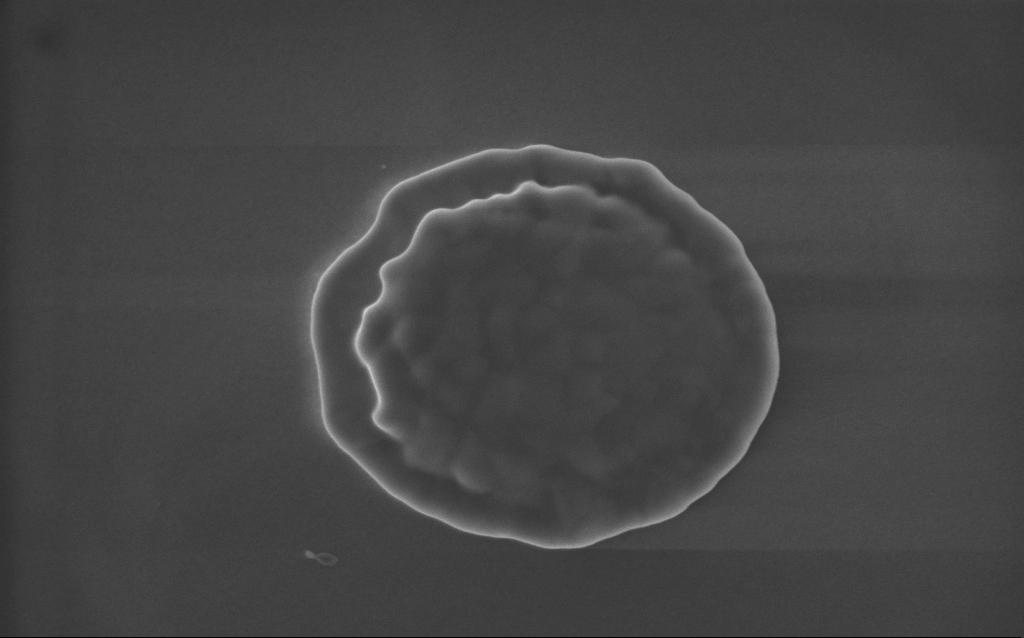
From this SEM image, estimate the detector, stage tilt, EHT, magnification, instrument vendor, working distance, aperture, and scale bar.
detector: InLens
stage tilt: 0°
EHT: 5 kV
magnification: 89 K X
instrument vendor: Zeiss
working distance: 3 mm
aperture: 30 µm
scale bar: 200 nm